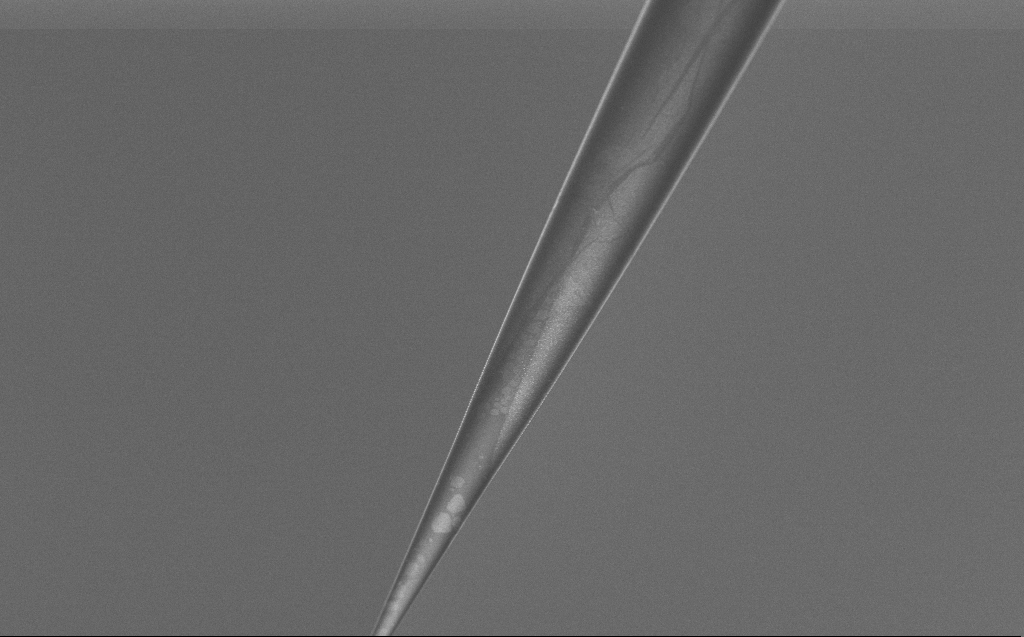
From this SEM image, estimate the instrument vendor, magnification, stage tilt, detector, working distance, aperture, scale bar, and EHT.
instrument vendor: Zeiss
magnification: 2.5 K X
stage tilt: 45°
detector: InLens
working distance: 6 mm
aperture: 30 µm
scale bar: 20000 nm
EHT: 5 kV